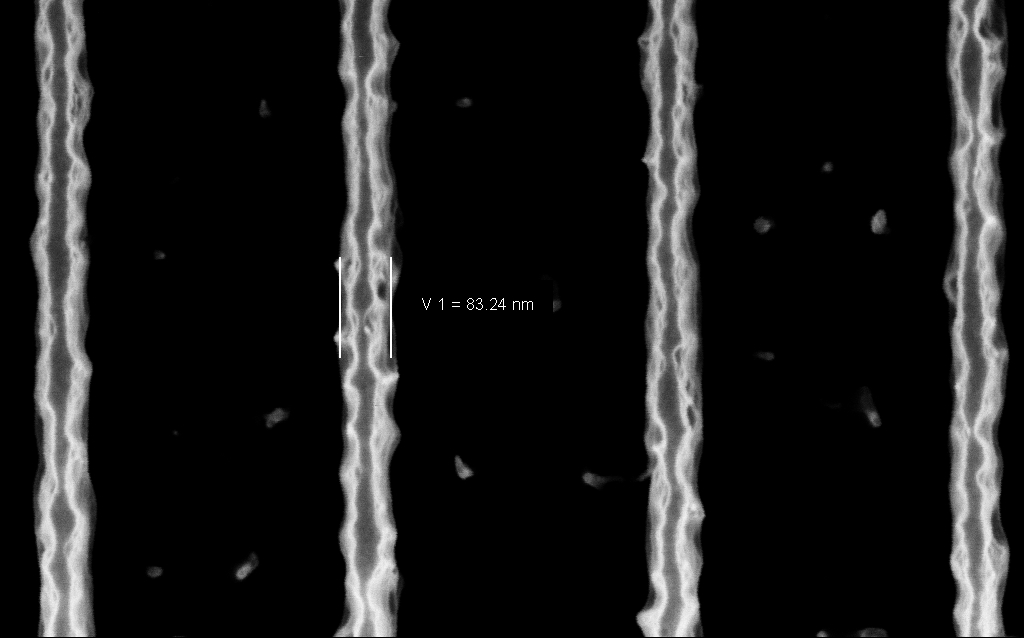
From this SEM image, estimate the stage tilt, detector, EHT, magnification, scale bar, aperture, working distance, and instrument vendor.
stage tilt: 0°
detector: InLens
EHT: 3 kV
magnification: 224.96 K X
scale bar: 200 nm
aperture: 30 µm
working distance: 5 mm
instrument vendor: Zeiss